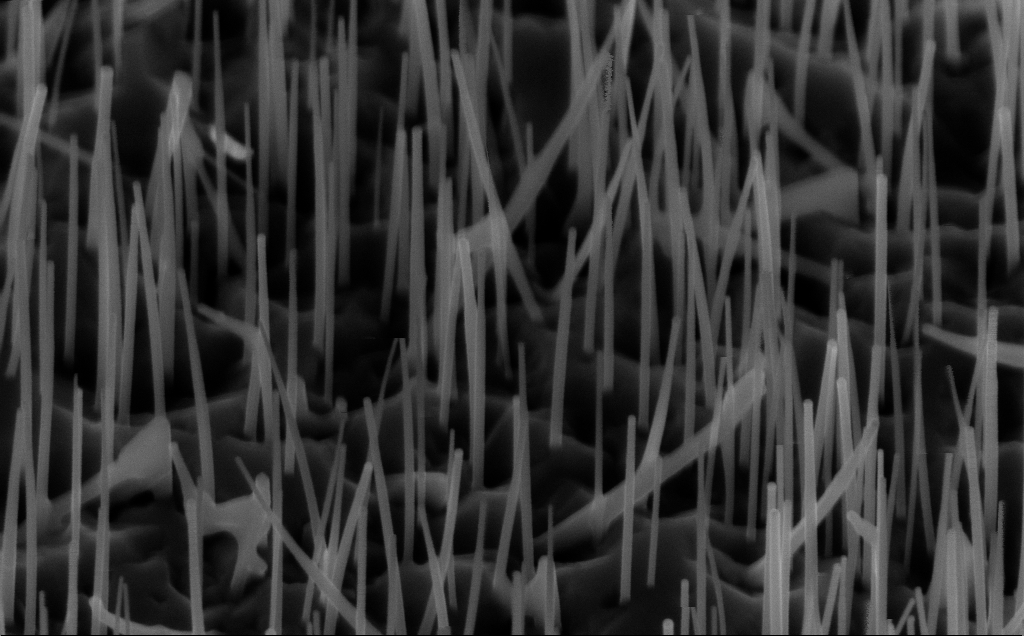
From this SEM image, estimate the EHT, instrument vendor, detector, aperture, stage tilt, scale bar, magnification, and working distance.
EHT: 10 kV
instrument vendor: Zeiss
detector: InLens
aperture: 30 µm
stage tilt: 45°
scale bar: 200 nm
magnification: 80 K X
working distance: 5 mm